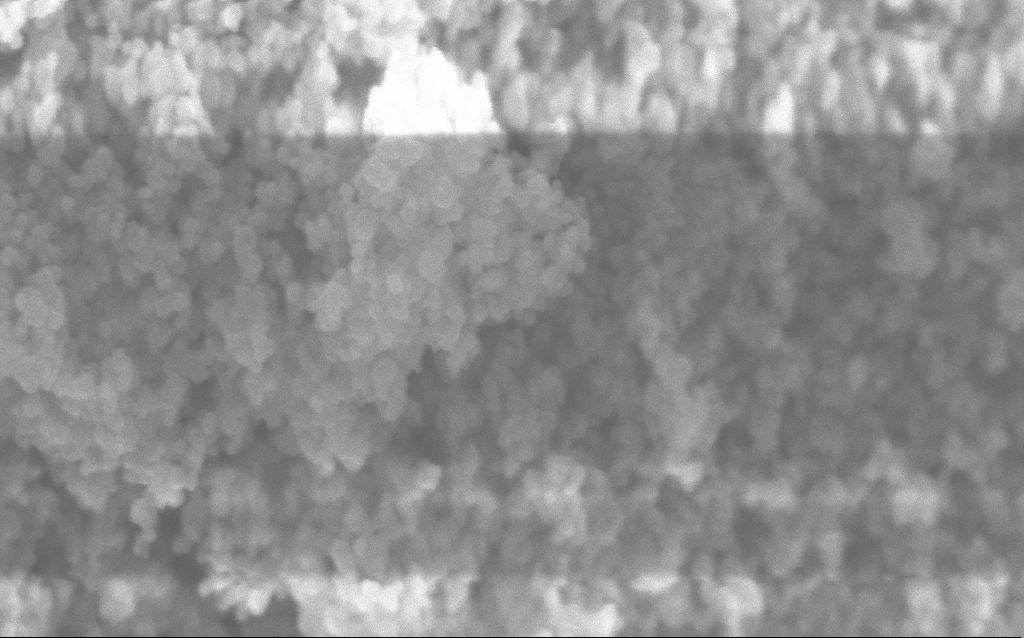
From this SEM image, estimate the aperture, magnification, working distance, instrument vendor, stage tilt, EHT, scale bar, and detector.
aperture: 30 µm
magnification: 211.33 K X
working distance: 2.5 mm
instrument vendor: Zeiss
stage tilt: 0°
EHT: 5 kV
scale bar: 100 nm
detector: InLens